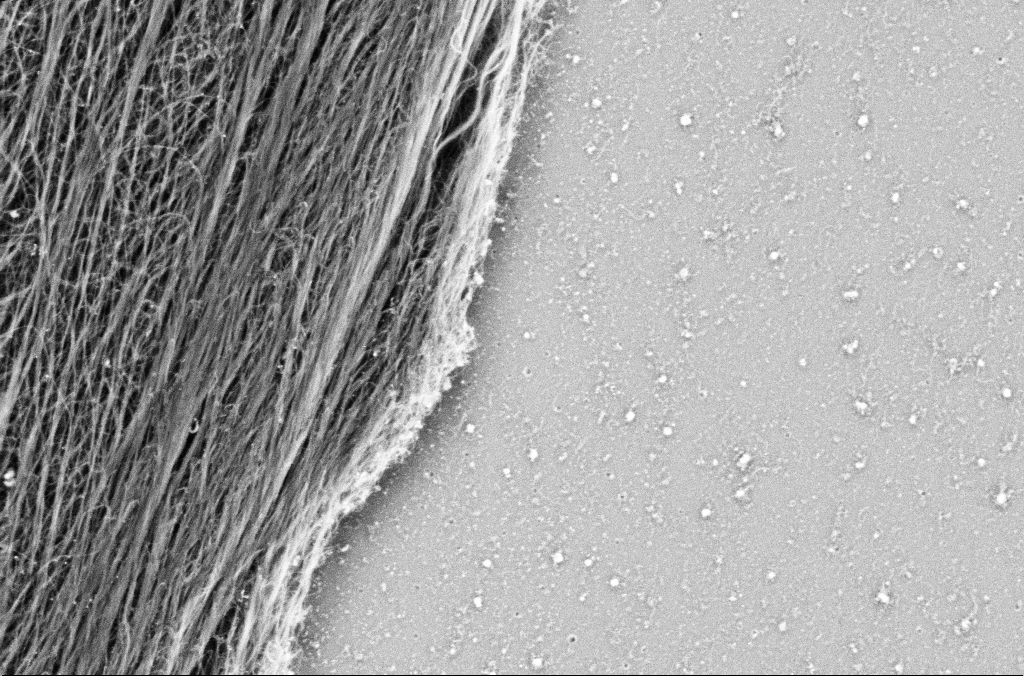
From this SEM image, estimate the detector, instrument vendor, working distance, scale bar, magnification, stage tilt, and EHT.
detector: SE2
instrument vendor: Zeiss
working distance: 5.7 mm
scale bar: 1000 nm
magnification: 50 K X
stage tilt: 0°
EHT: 1.8 kV